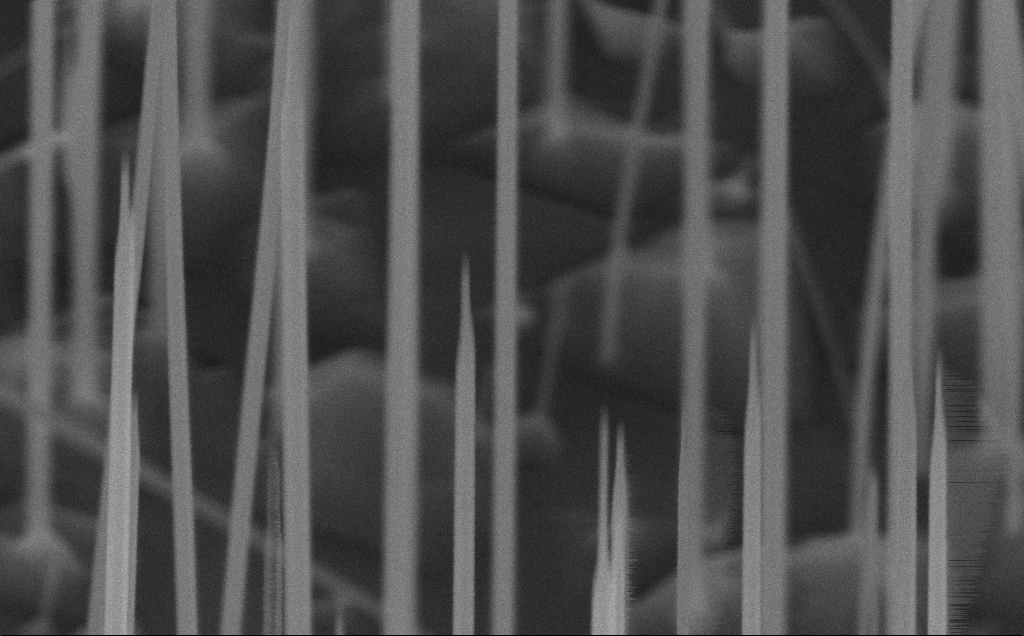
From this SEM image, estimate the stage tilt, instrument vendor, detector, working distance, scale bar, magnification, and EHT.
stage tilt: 45°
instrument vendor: Zeiss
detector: InLens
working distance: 8 mm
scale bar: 200 nm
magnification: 135.95 K X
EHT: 10 kV